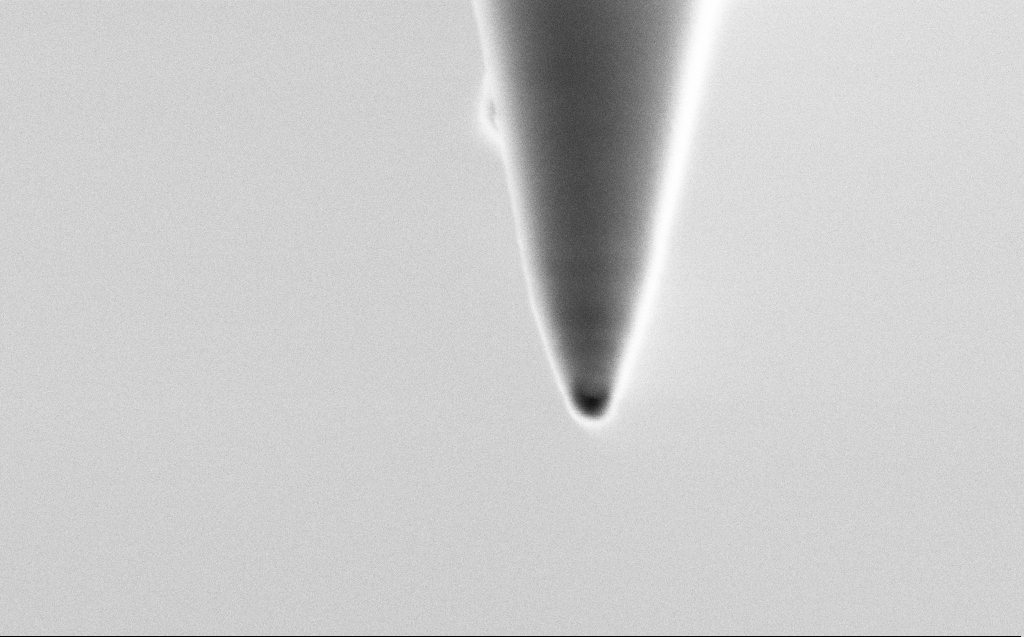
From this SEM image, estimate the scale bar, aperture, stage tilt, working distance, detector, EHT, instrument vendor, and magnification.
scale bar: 200 nm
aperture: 30 µm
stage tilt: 45.1°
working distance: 3 mm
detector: SE2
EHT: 0.75 kV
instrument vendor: Zeiss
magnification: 161.01 K X